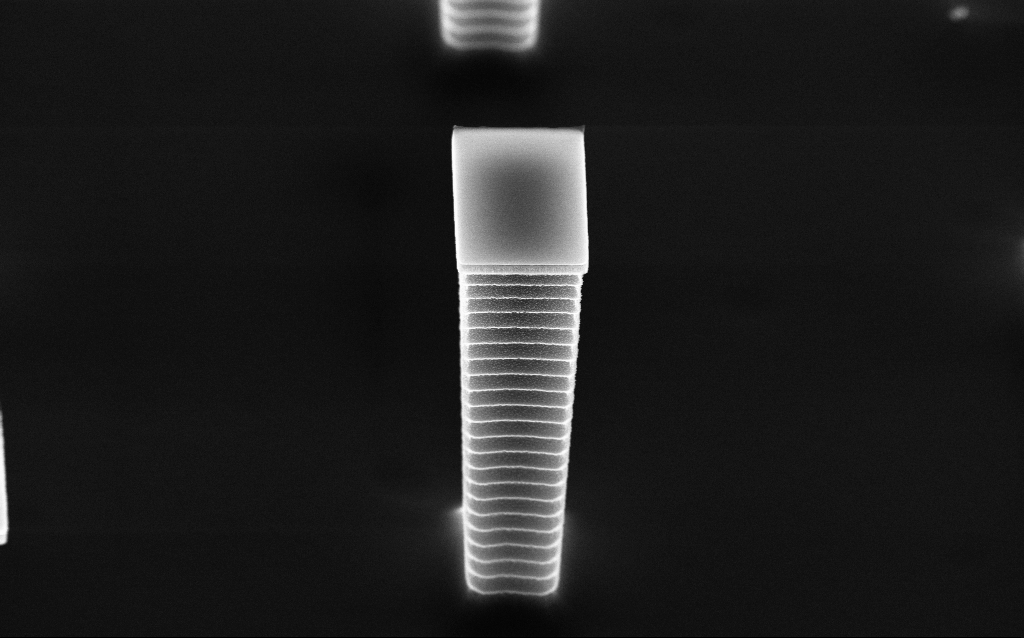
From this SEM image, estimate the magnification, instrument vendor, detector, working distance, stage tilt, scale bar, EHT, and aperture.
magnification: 24.01 K X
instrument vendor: Zeiss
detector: InLens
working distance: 4.8 mm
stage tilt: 45°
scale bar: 2000 nm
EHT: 10 kV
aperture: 30 µm